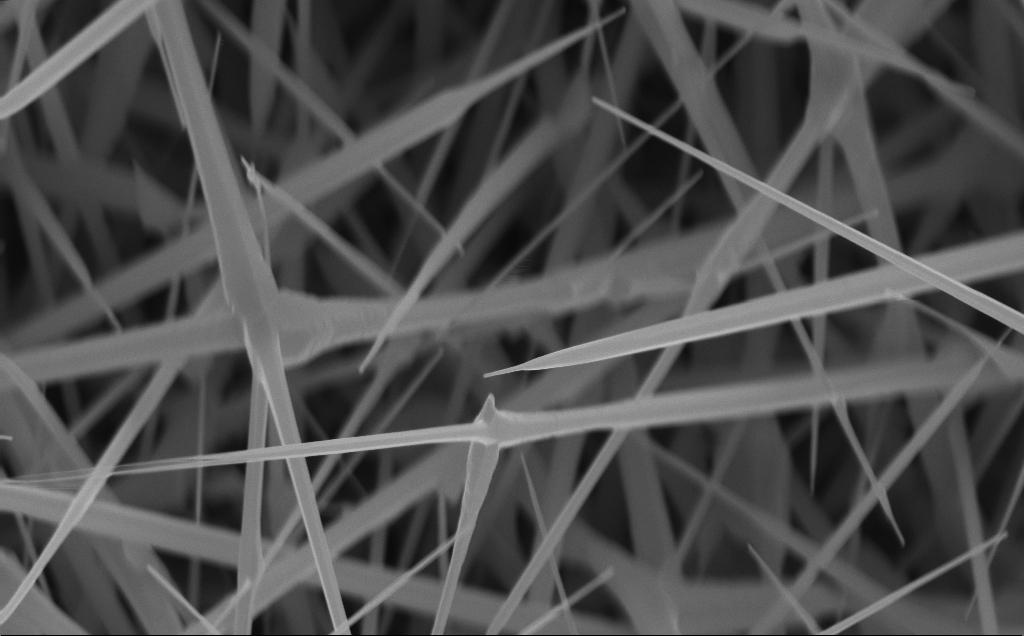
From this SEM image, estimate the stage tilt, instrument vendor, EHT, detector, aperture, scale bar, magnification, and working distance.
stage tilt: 0°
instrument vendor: Zeiss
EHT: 10 kV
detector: InLens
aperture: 30 µm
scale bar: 1000 nm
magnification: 40 K X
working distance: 4 mm